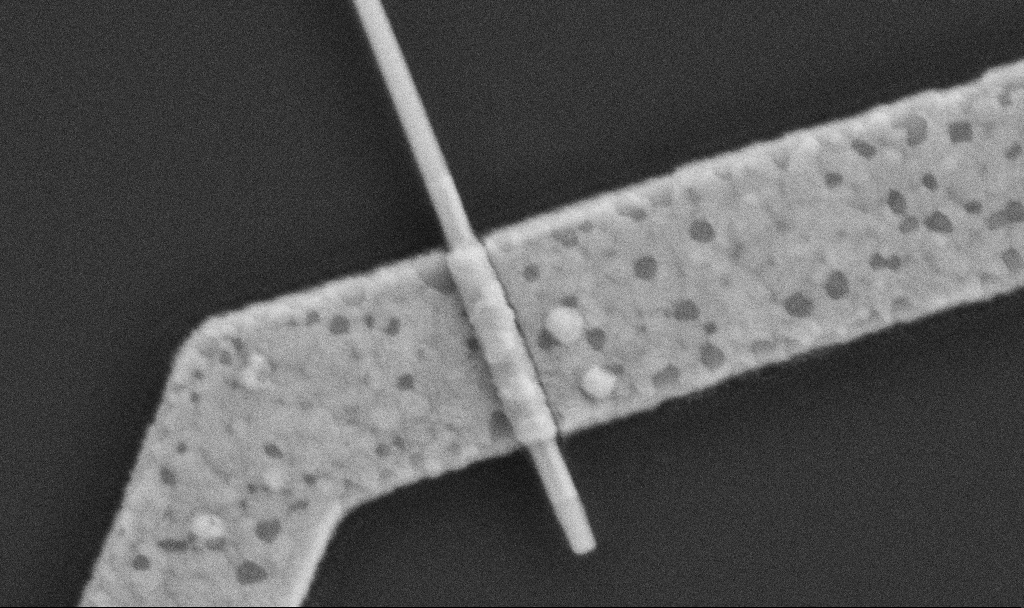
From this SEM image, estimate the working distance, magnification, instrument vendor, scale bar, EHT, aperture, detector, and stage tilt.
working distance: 7.6 mm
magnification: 100 K X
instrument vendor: Zeiss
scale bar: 200 nm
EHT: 5 kV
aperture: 30 µm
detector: SE2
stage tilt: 0°